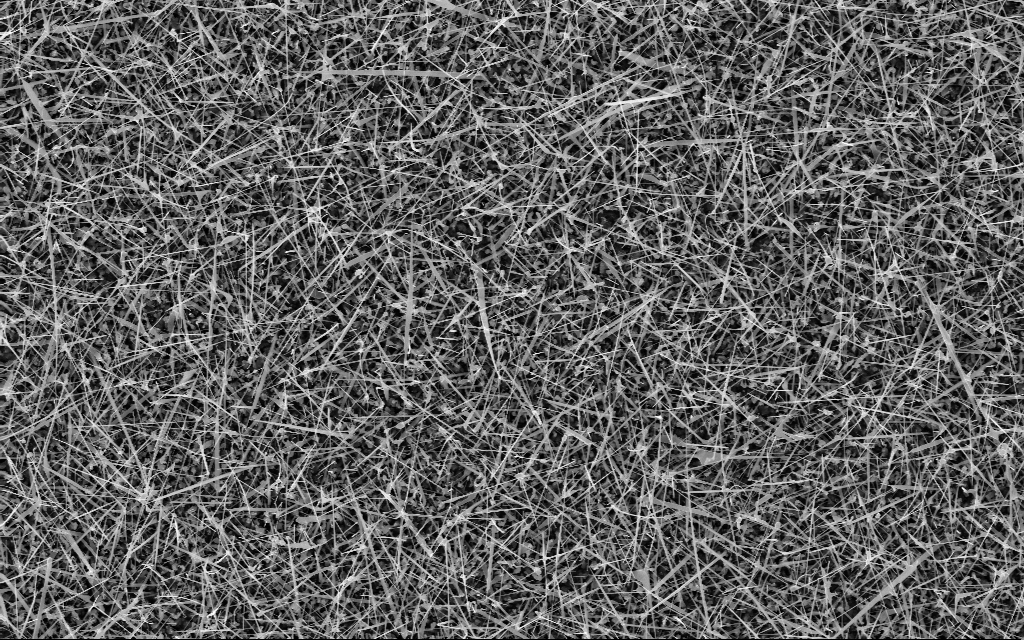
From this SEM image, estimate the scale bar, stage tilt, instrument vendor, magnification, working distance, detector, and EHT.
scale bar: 2000 nm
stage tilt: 0°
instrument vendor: Zeiss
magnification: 10 K X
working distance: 7 mm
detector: InLens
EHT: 10 kV